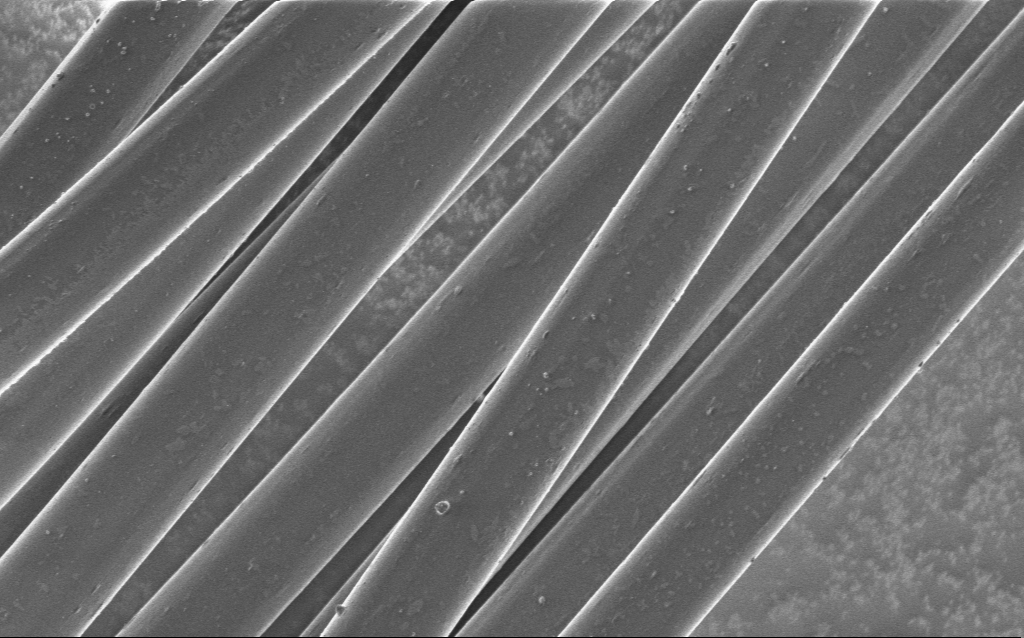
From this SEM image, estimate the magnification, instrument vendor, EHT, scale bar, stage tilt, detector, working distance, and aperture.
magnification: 2.88 K X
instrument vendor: Zeiss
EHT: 1 kV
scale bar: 20000 nm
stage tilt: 0°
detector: InLens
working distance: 5 mm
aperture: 30 µm